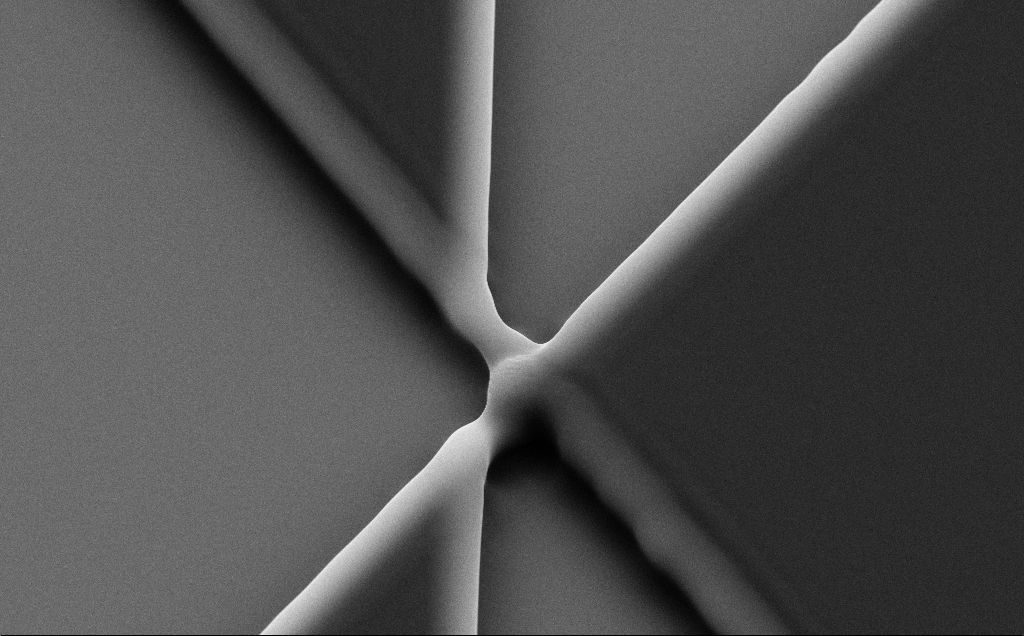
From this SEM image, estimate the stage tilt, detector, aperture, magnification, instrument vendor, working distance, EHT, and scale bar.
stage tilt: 35°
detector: SE2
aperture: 30 µm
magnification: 12.97 K X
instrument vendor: Zeiss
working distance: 8 mm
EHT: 10 kV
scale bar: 1000 nm